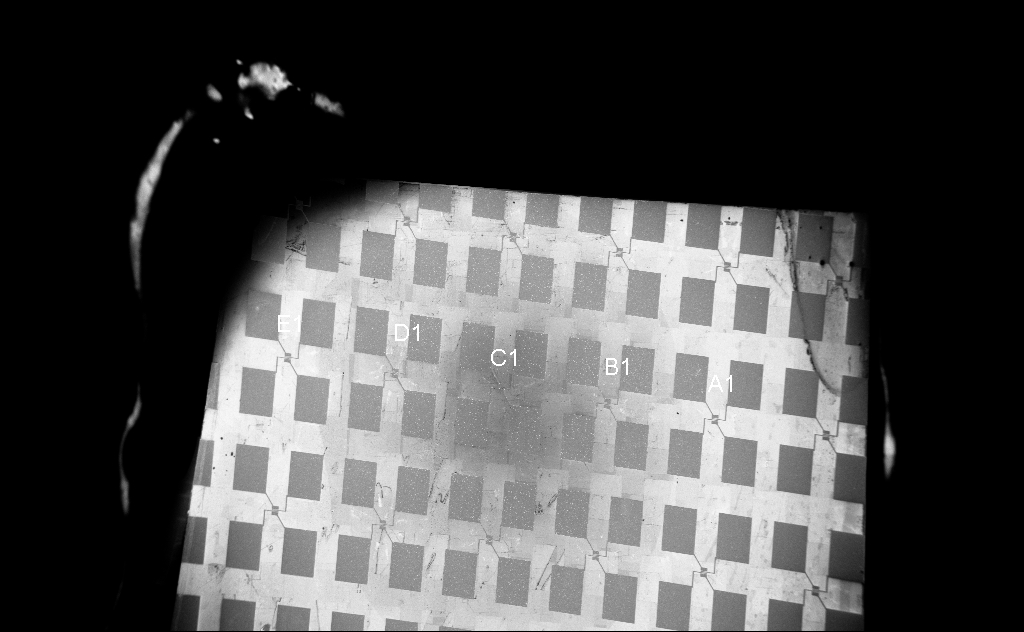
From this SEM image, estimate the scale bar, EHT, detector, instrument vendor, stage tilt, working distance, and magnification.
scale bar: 200000 nm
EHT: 5 kV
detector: InLens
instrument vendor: Zeiss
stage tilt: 0°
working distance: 6 mm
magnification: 0.08 K X